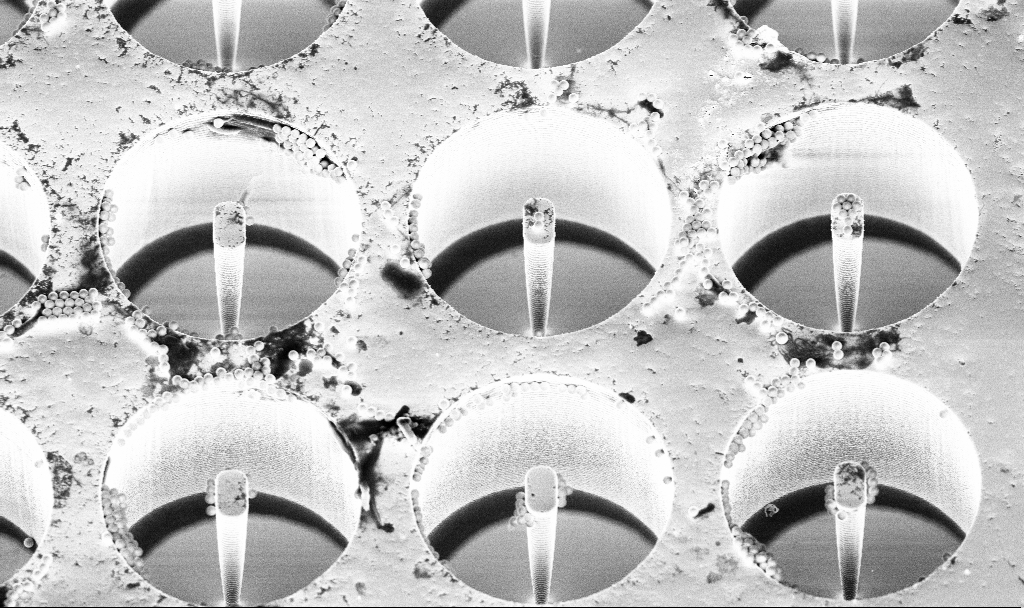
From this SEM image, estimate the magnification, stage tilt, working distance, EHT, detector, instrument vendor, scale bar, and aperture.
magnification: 4.94 K X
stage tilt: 30°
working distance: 7.1 mm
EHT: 5 kV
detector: InLens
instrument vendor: Zeiss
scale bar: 10000 nm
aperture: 30 µm